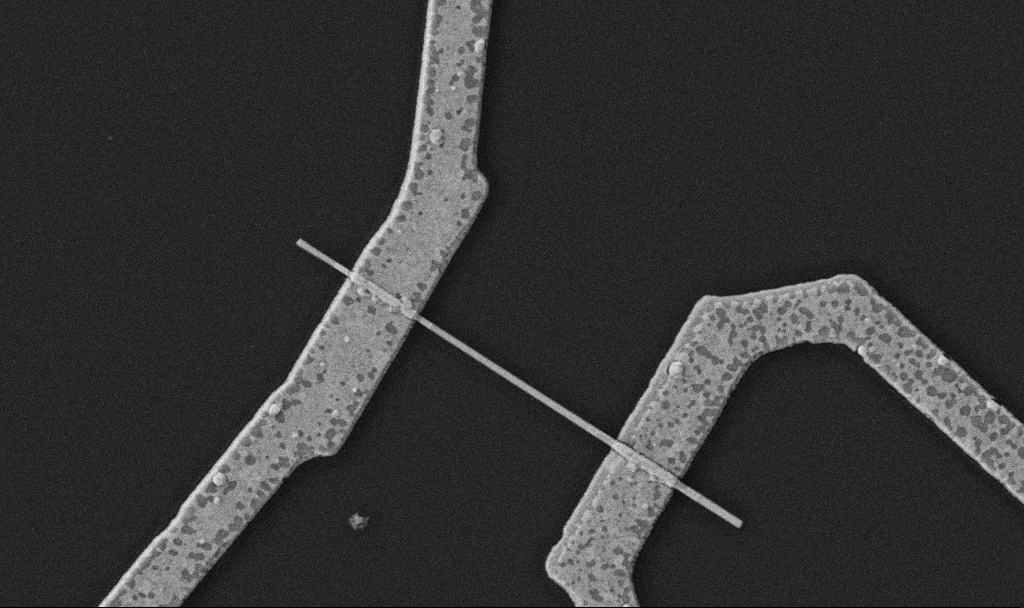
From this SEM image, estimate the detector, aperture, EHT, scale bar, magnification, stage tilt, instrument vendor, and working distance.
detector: SE2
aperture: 30 µm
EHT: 5 kV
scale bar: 1000 nm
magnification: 30 K X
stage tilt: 0°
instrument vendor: Zeiss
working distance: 10.7 mm